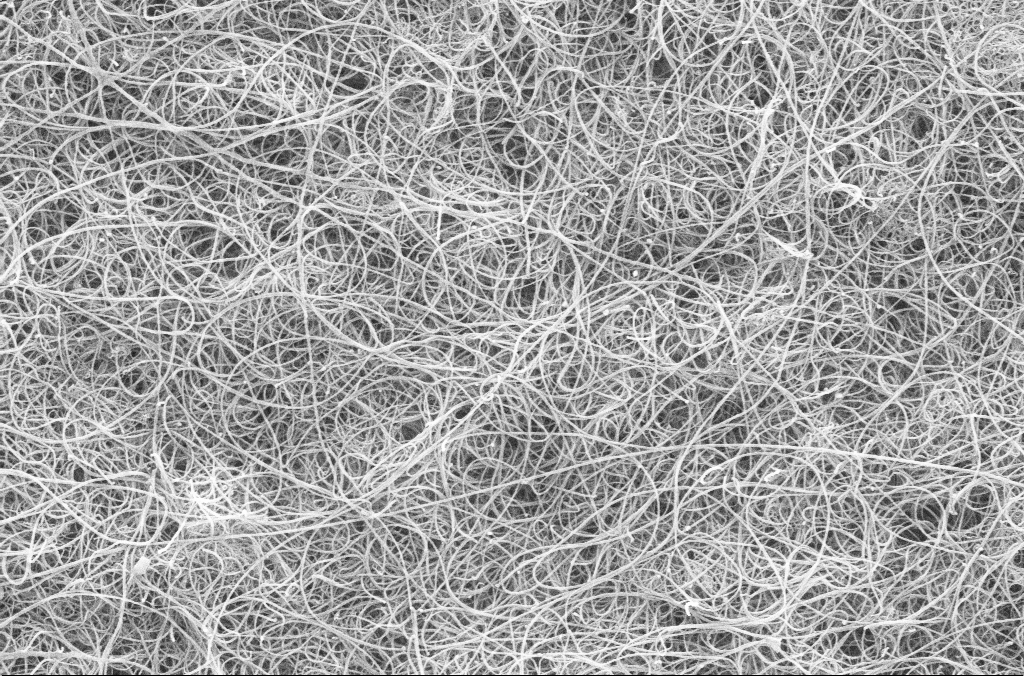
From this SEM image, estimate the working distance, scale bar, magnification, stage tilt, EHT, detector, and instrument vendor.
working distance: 3 mm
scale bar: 2000 nm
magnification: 25 K X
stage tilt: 0°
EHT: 5 kV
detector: SE2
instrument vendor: Zeiss